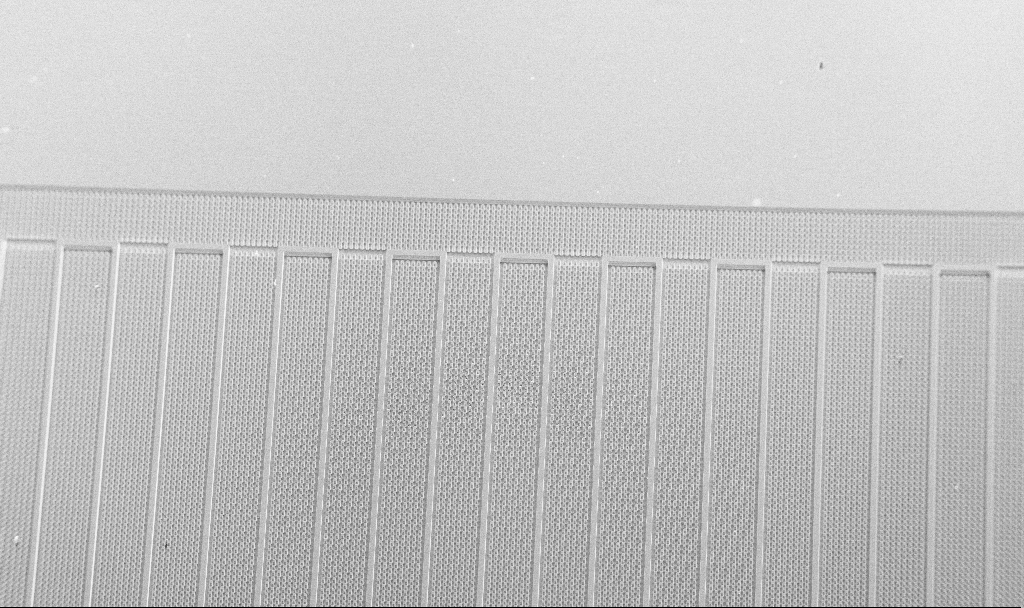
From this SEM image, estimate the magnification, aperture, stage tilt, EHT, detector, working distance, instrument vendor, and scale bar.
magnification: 0.099 K X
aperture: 30 µm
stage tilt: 45°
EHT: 5 kV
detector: SE2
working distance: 16.6 mm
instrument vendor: Zeiss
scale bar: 200000 nm